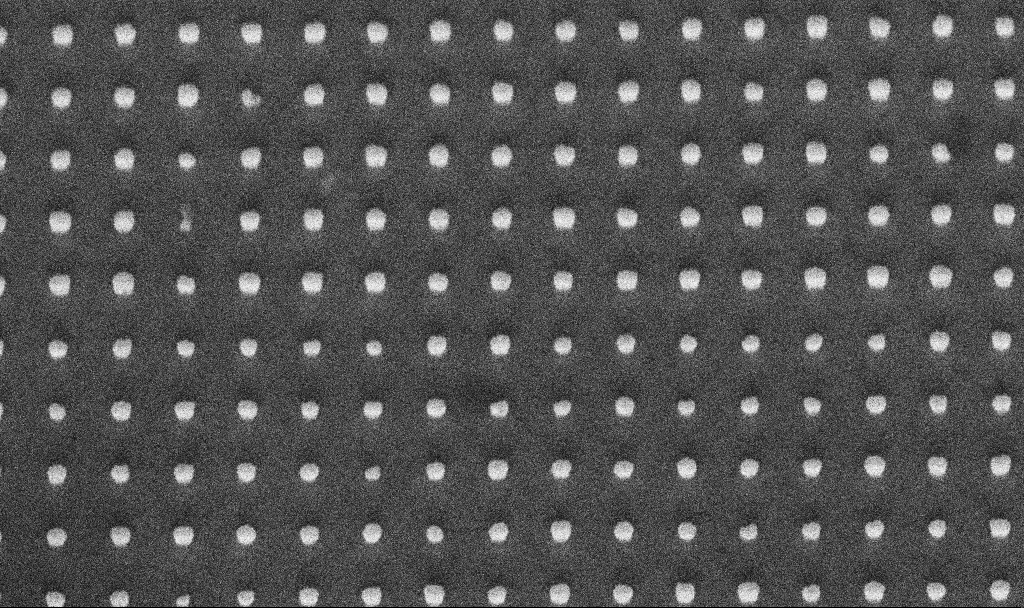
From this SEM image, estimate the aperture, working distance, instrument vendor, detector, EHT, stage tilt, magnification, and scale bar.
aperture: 30 µm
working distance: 8.8 mm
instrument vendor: Zeiss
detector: InLens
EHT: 5 kV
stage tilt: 45°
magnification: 76.65 K X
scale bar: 200 nm